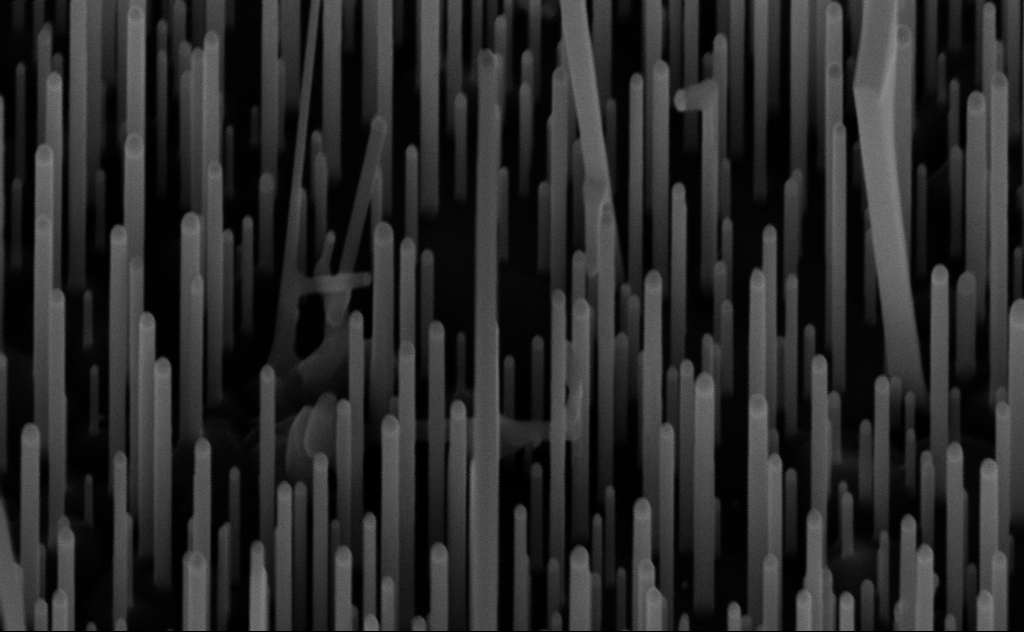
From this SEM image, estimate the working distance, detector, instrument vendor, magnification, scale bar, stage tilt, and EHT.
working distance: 7 mm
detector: InLens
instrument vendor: Zeiss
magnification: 100 K X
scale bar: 200 nm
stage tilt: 45°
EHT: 10 kV